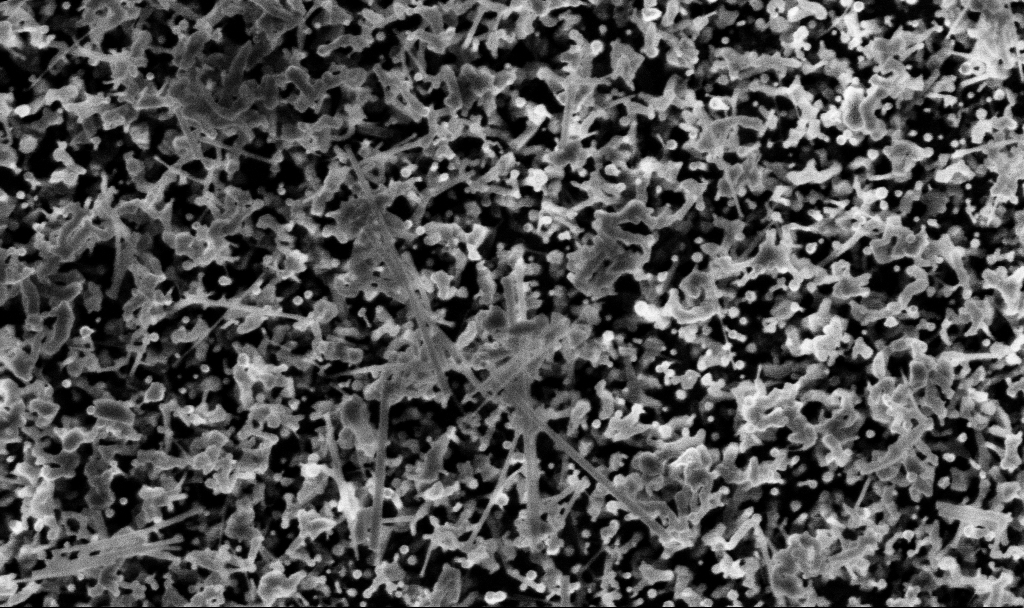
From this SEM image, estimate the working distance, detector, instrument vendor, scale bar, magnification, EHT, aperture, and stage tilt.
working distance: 3.1 mm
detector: InLens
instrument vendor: Zeiss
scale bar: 1000 nm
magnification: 67.29 K X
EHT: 3 kV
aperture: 30 µm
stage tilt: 0°